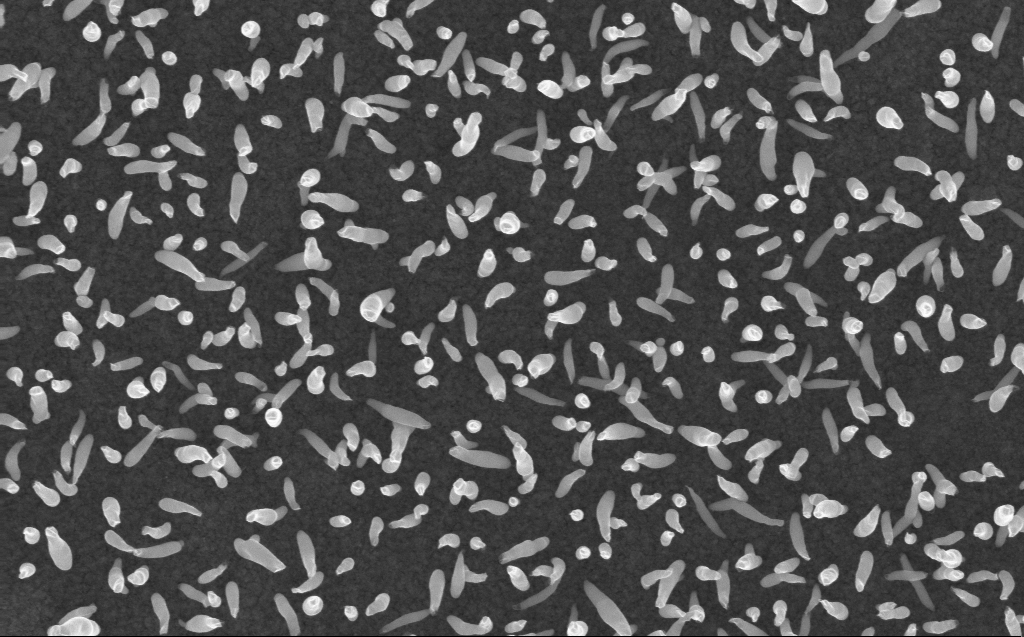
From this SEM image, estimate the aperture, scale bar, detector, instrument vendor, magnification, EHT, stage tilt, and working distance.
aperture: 30 µm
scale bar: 1000 nm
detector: InLens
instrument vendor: Zeiss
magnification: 50 K X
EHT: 10 kV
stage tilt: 0°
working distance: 3 mm